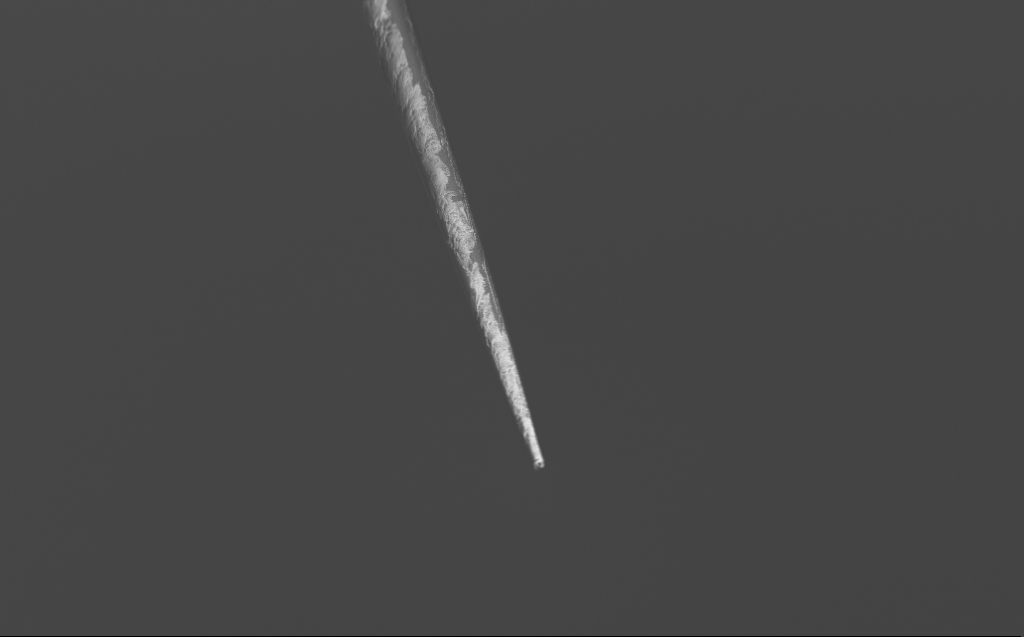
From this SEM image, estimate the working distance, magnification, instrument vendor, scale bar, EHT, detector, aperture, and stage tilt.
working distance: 4 mm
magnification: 0.744 K X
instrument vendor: Zeiss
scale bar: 20000 nm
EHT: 3 kV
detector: InLens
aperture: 30 µm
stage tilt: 45°